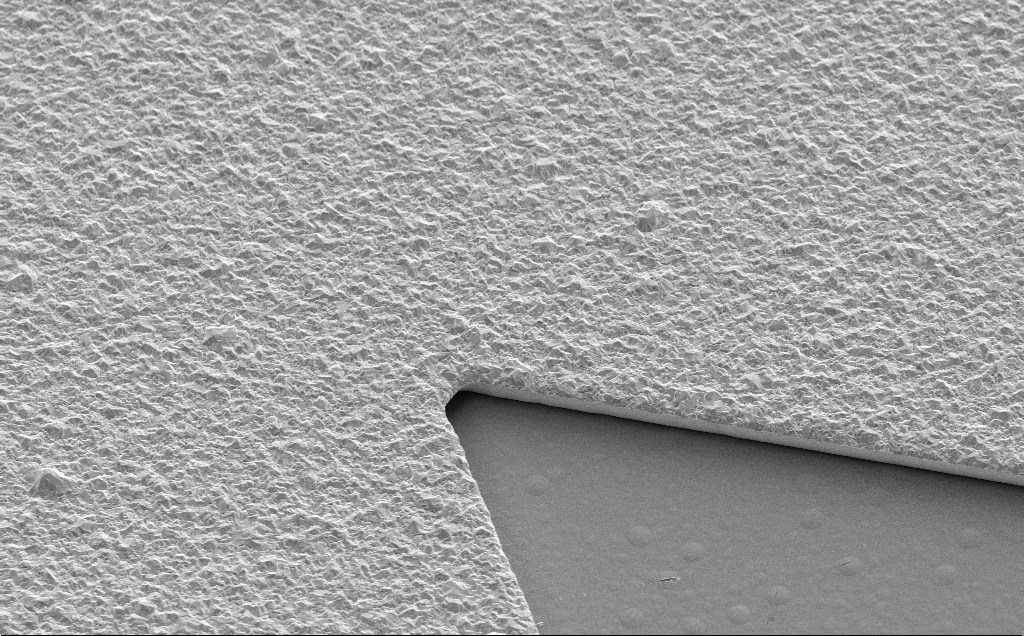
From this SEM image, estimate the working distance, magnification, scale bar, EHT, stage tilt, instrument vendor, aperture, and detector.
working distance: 13 mm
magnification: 7.78 K X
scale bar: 2000 nm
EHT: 5 kV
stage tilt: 35°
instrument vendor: Zeiss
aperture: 30 µm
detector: SE2